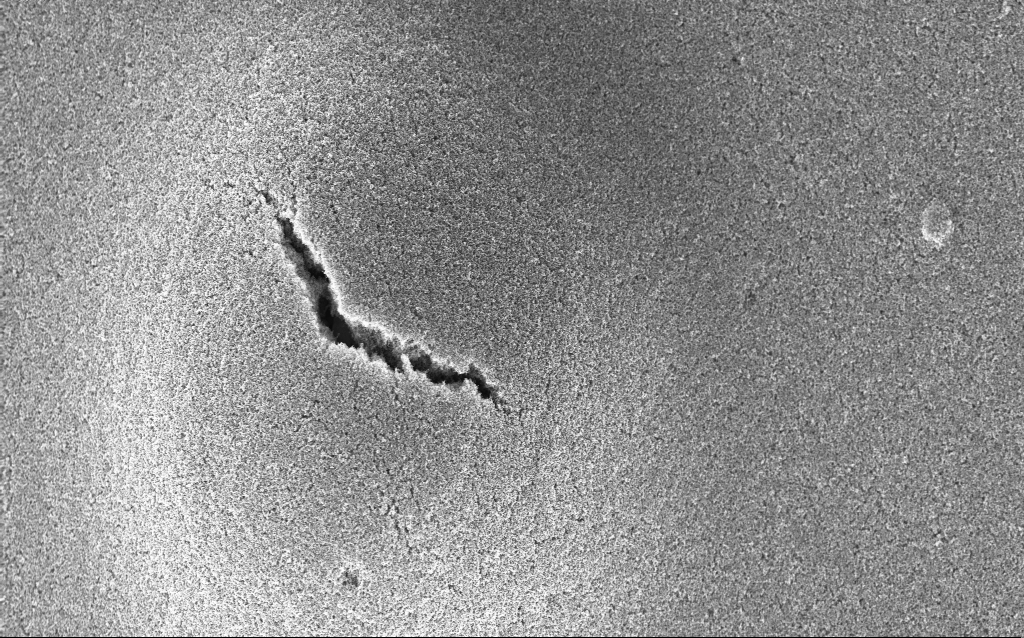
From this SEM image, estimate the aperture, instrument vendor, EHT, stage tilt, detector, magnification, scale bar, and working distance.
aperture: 30 µm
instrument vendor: Zeiss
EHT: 5 kV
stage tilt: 0°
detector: InLens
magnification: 15.33 K X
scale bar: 1000 nm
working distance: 2.6 mm